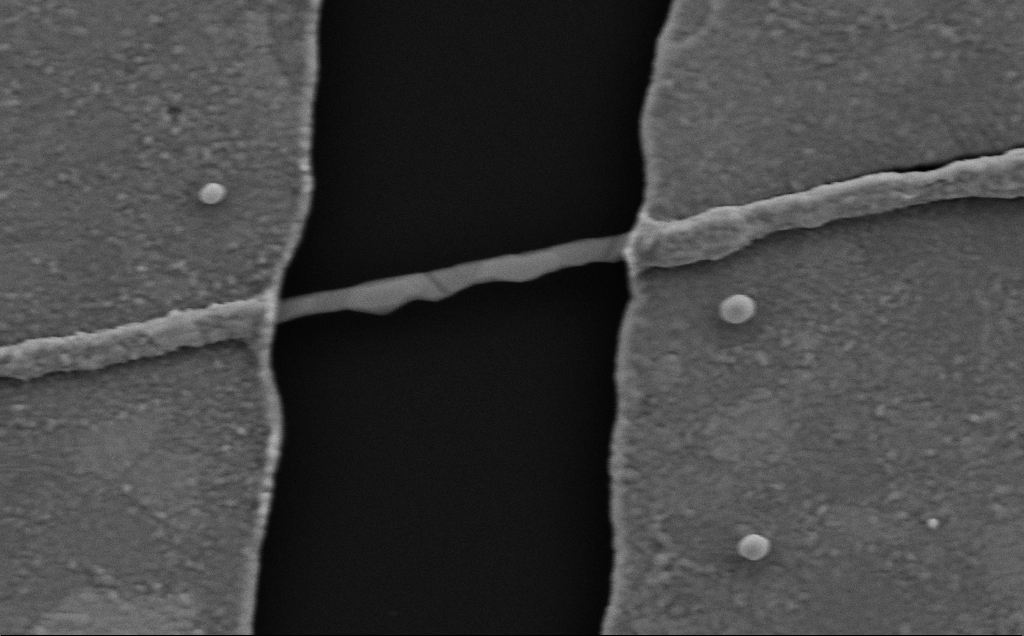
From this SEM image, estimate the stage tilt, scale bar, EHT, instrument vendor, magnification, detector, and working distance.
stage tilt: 0°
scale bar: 200 nm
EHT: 5 kV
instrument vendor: Zeiss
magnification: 83.12 K X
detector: SE2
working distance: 6 mm